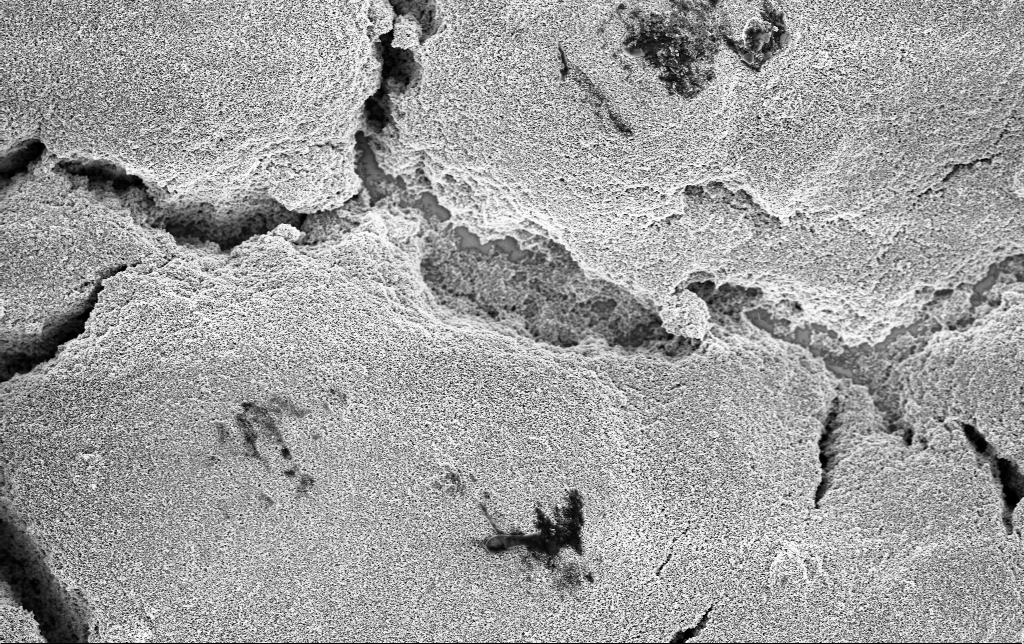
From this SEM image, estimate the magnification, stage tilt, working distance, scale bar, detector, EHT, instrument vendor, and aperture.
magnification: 5 K X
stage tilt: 0°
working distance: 2.8 mm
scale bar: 10000 nm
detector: InLens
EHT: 3 kV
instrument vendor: Zeiss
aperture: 30 µm